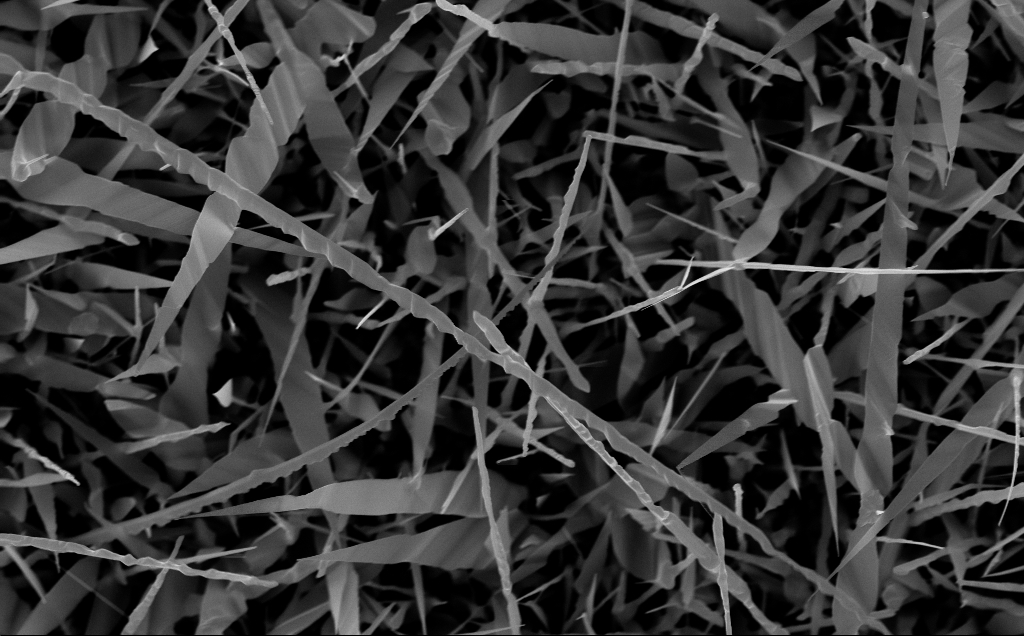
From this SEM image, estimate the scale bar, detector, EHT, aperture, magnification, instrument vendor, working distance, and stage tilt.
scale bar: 2000 nm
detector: InLens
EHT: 10 kV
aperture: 30 µm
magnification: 20 K X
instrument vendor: Zeiss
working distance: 6 mm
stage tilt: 0°